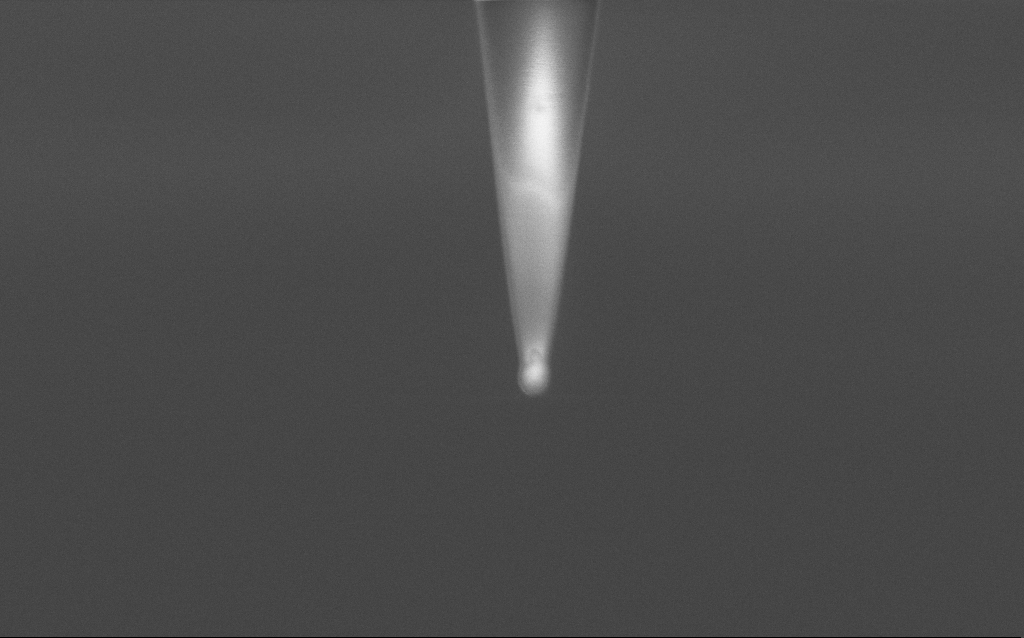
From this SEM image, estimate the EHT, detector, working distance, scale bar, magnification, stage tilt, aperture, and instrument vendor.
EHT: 2 kV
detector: InLens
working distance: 6.7 mm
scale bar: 1000 nm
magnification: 70 K X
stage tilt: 44.9°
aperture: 30 µm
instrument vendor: Zeiss